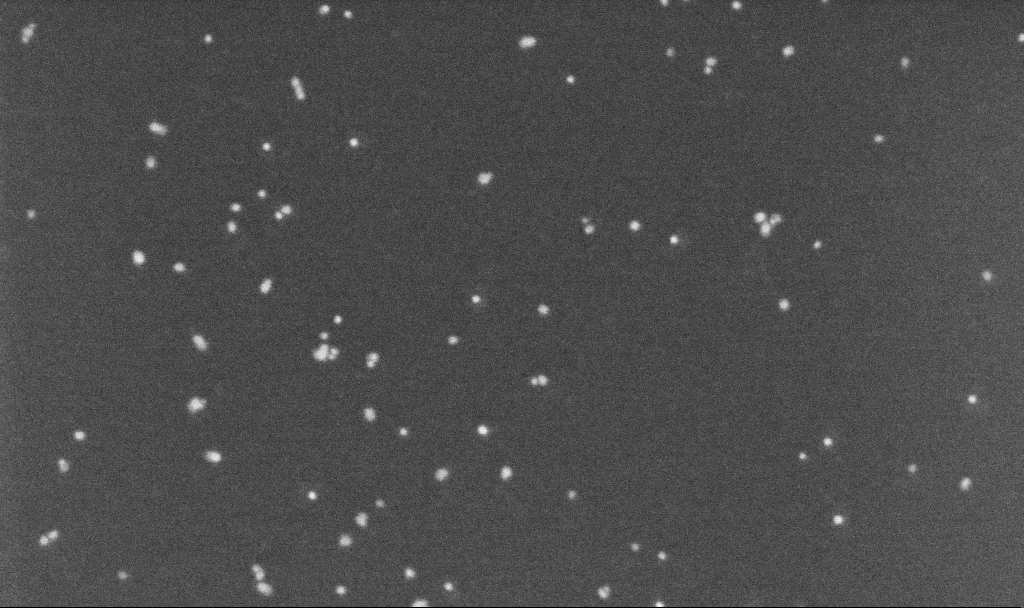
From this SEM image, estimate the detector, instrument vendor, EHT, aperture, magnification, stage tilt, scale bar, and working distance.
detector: InLens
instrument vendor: Zeiss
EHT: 5 kV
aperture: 30 µm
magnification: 250 K X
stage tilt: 0°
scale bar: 100 nm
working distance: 3.2 mm